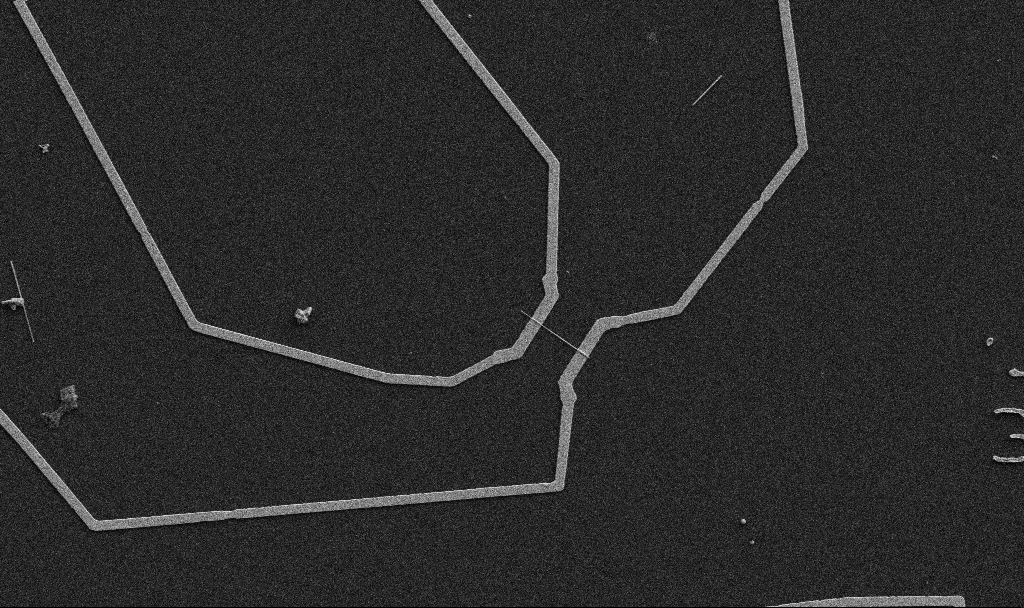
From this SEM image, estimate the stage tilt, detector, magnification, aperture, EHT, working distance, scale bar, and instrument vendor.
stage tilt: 0°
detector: SE2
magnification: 5 K X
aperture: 30 µm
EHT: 5 kV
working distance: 10.7 mm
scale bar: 10000 nm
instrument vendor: Zeiss